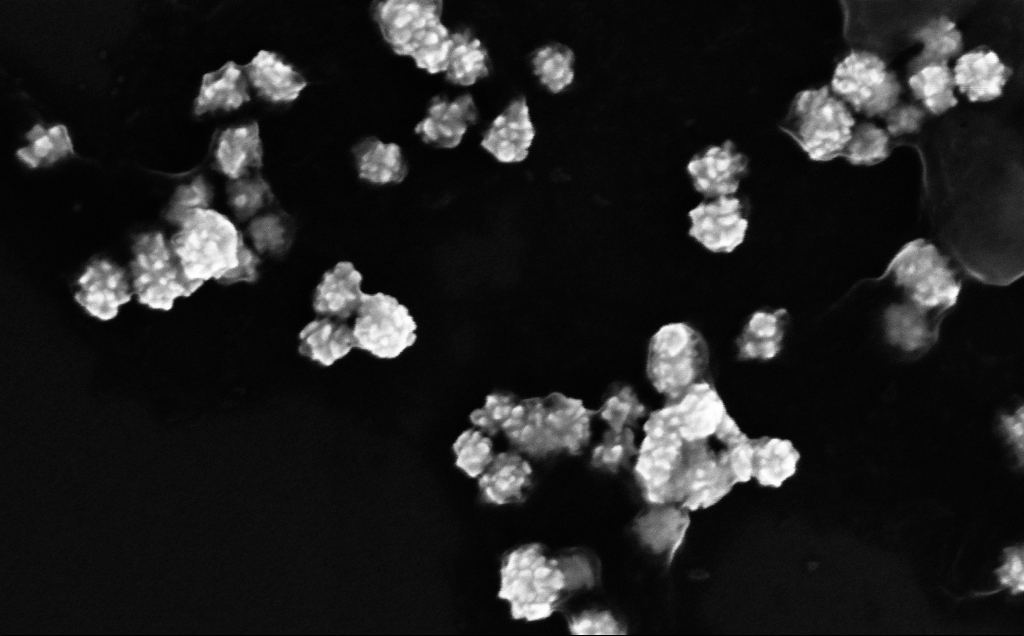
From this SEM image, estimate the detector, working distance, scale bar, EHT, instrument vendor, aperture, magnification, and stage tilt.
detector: InLens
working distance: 4 mm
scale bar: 200 nm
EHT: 10 kV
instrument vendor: Zeiss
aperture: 30 µm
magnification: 292.35 K X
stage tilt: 0°